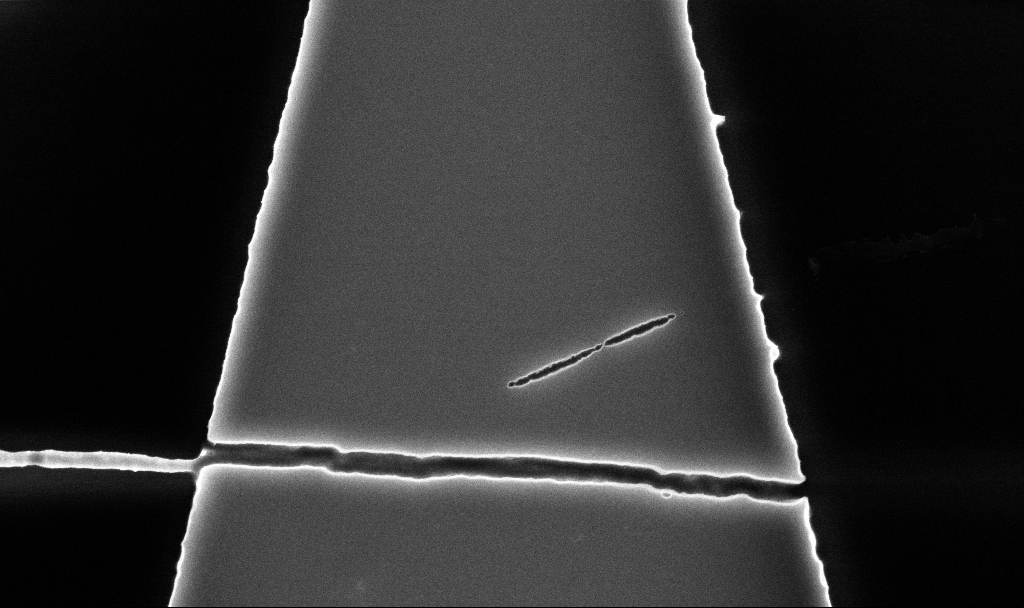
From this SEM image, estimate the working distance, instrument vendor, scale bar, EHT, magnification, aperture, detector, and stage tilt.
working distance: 5.2 mm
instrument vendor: Zeiss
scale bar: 1000 nm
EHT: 5 kV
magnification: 40.06 K X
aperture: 30 µm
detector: InLens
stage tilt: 0°